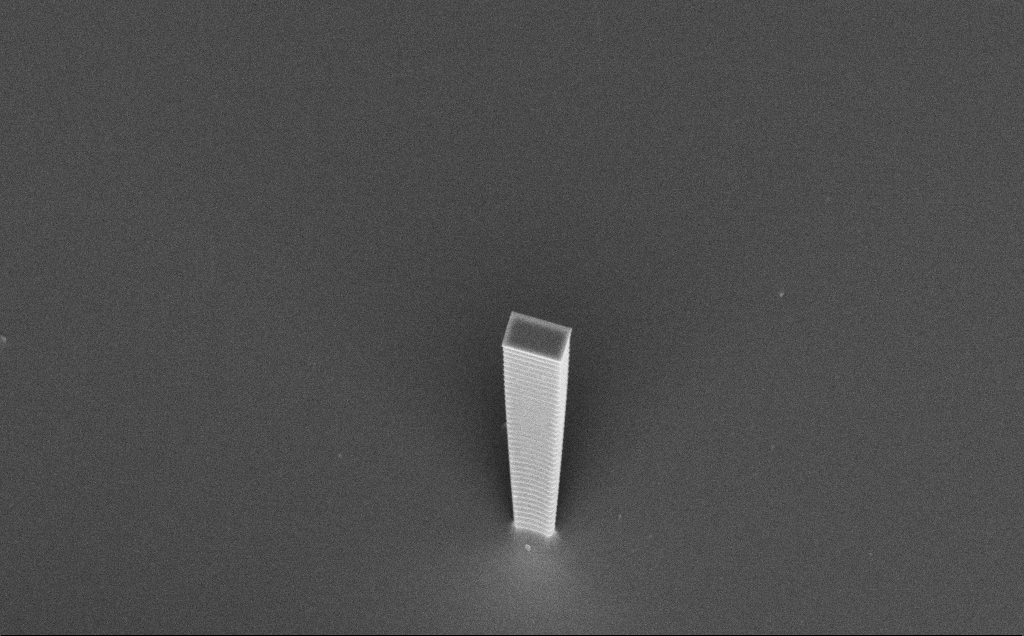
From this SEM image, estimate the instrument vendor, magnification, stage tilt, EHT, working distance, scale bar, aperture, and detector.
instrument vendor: Zeiss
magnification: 4.8 K X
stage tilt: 45°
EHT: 7.5 kV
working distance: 8 mm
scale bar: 10000 nm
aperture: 30 µm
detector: InLens